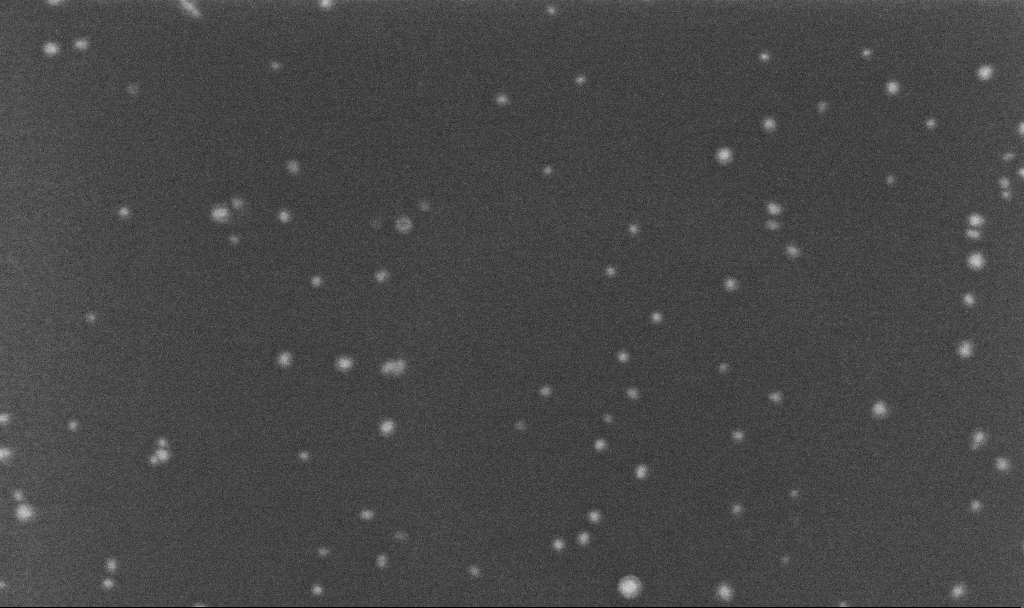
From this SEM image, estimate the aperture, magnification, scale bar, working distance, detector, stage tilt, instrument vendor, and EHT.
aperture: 30 µm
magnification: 300 K X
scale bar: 100 nm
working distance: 3.2 mm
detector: InLens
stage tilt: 0°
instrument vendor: Zeiss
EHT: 5 kV